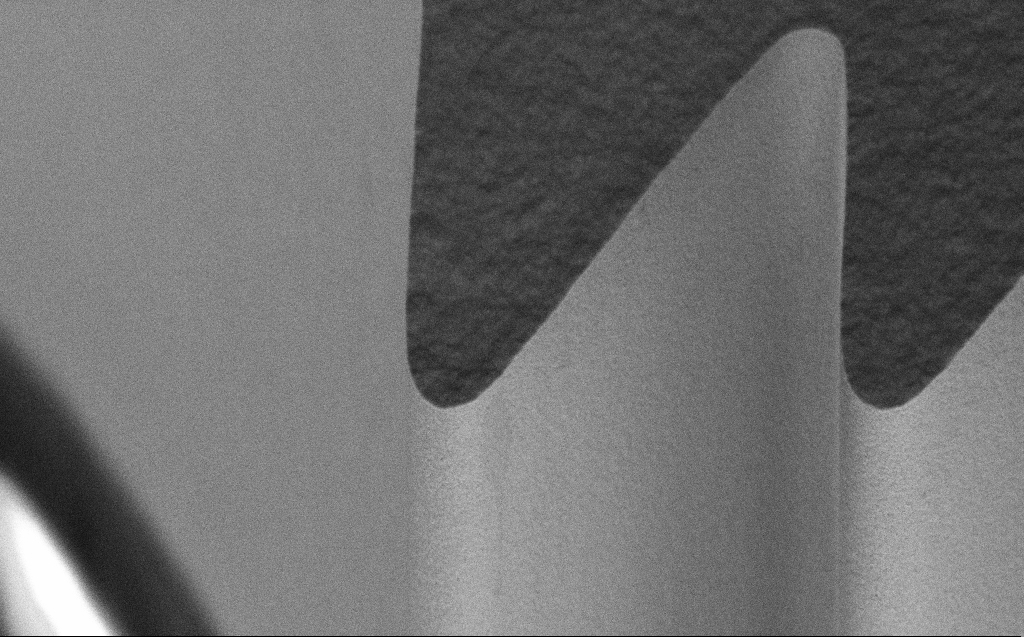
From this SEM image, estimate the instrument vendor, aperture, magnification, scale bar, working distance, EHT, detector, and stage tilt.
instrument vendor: Zeiss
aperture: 30 µm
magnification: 14.16 K X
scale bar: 1000 nm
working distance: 7 mm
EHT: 5 kV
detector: InLens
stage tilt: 45°